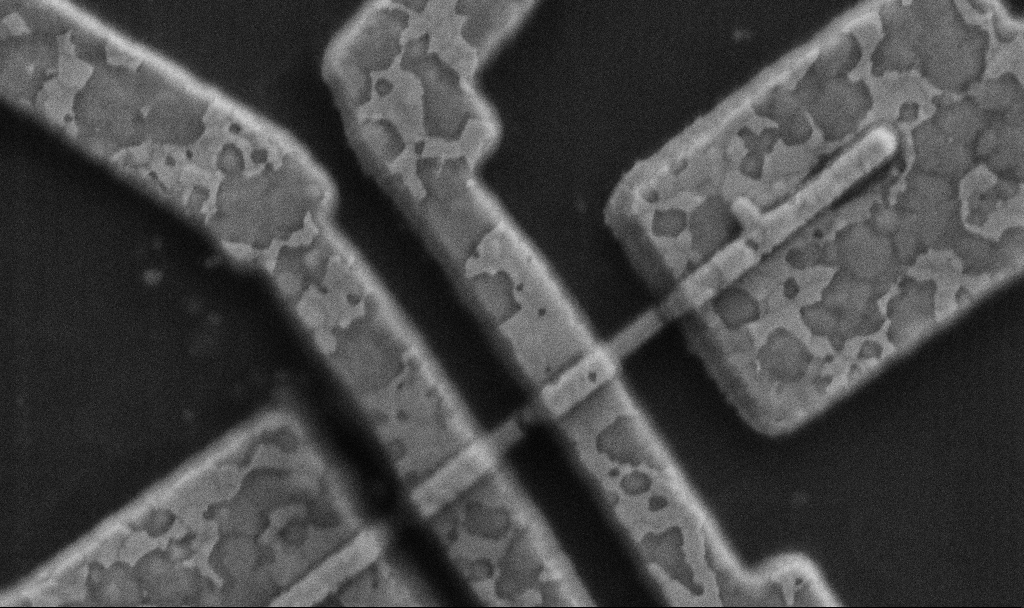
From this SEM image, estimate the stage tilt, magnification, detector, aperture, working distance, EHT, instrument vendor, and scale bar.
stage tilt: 0°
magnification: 50 K X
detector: SE2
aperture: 30 µm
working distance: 8.5 mm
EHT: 5 kV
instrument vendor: Zeiss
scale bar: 1000 nm